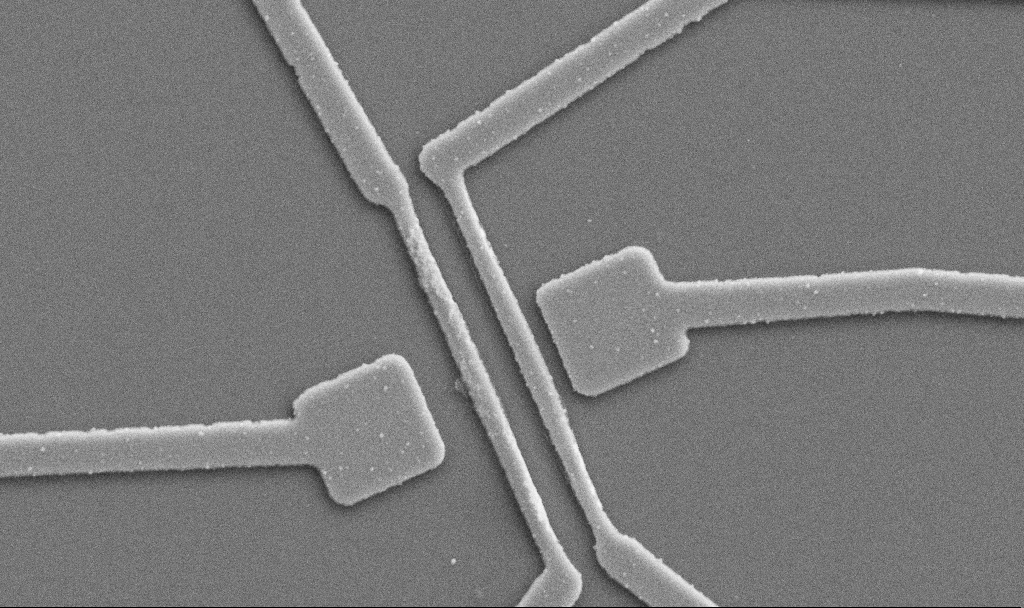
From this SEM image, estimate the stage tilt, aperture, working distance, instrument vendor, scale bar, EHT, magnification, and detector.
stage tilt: -0°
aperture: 30 µm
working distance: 10.7 mm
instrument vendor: Zeiss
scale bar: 1000 nm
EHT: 5 kV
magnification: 20 K X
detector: SE2